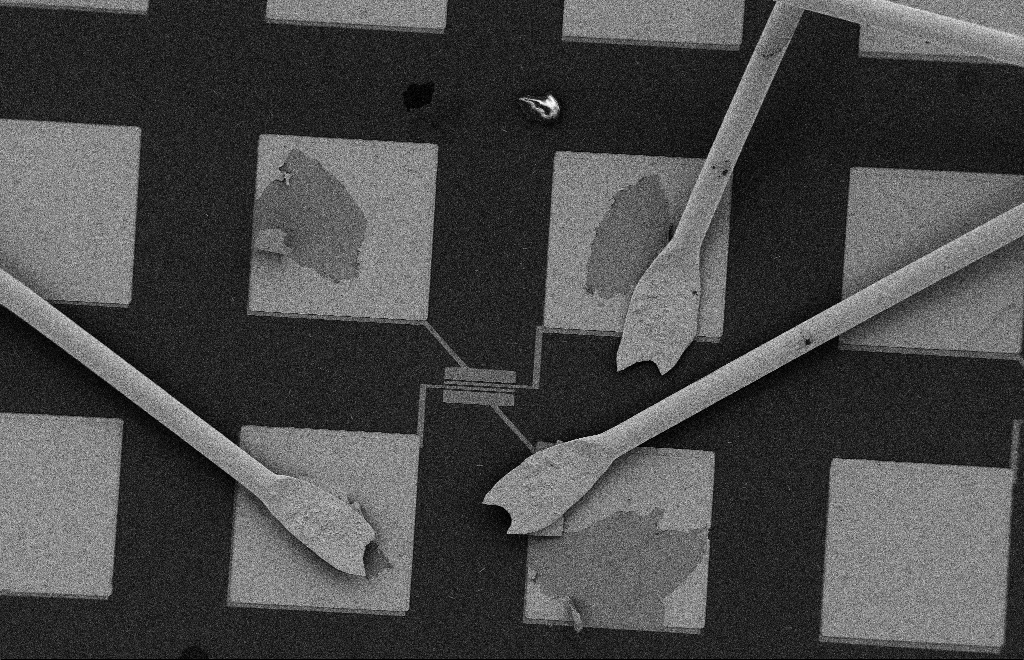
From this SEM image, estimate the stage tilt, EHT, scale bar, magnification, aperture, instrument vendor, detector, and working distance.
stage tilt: -0.3°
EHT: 2 kV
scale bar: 100000 nm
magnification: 0.428 K X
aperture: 20 µm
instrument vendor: Zeiss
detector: SE2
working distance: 10 mm